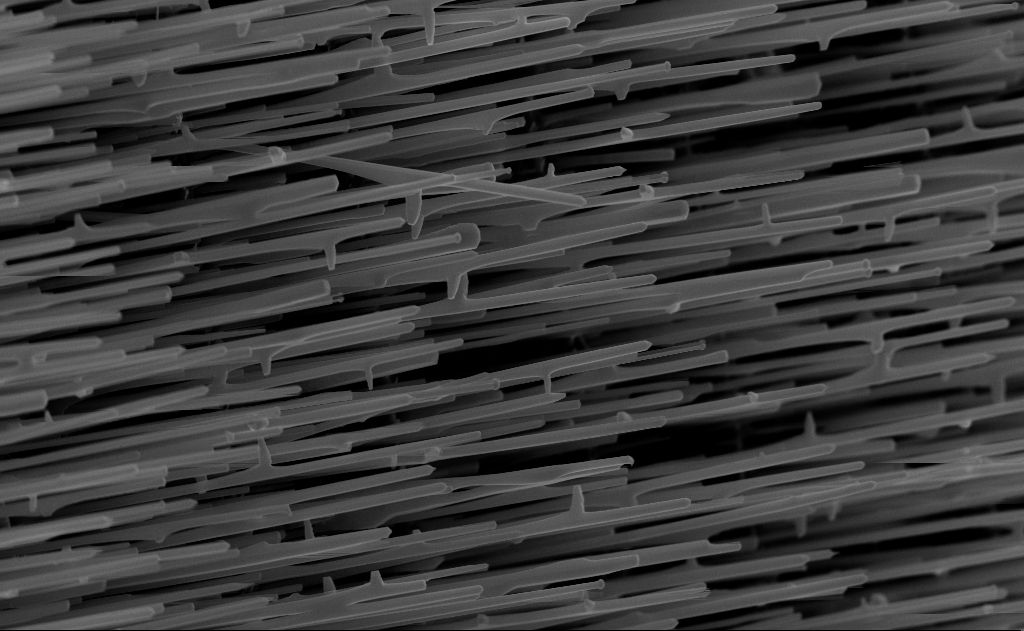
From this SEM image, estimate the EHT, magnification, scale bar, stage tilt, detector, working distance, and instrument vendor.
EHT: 10 kV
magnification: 20 K X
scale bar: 2000 nm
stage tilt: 0°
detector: InLens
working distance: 7 mm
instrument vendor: Zeiss